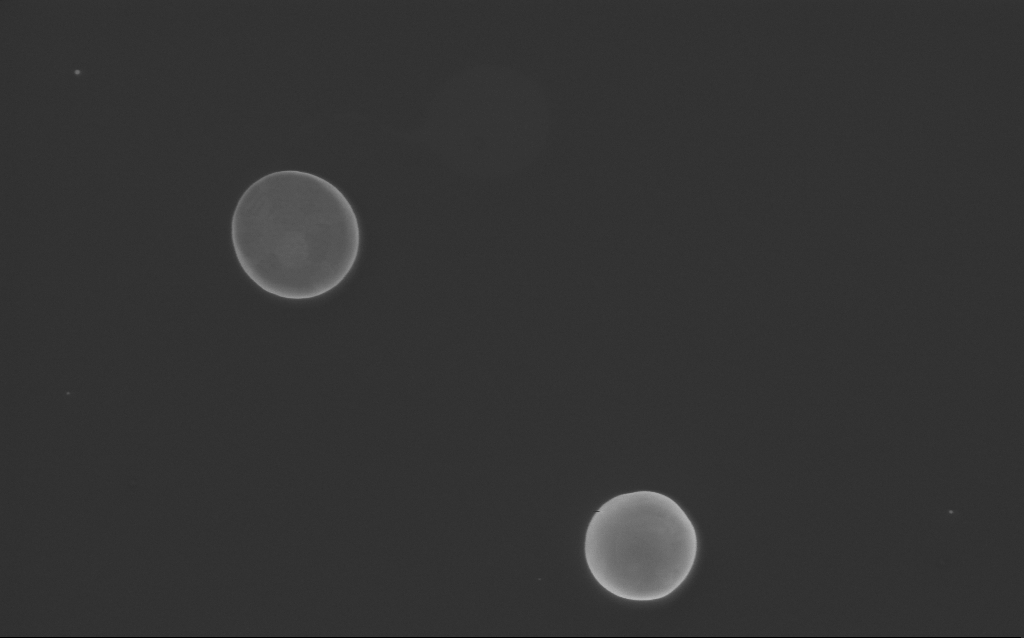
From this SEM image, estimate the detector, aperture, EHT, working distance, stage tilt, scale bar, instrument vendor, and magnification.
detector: InLens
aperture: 30 µm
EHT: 3 kV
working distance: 3 mm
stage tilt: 0°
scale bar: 1000 nm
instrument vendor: Zeiss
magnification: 63.71 K X